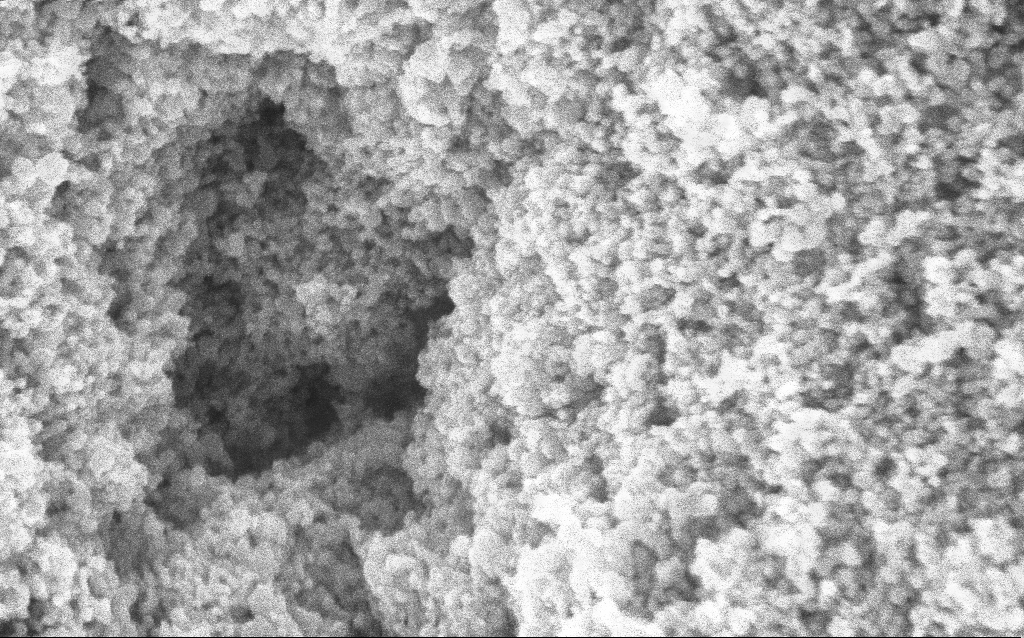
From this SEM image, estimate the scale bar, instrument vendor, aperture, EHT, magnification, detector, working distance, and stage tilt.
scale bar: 100 nm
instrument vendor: Zeiss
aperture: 30 µm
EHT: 5 kV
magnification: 162.39 K X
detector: InLens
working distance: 4.2 mm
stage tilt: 0°